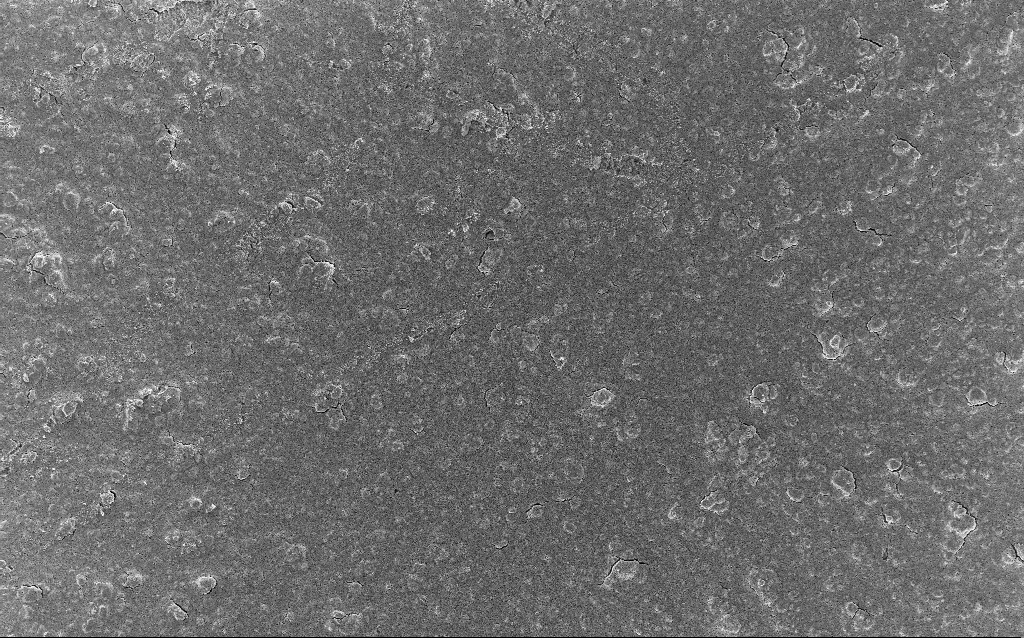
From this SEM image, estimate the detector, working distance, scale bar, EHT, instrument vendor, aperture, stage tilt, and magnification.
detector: InLens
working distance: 2.9 mm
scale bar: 20000 nm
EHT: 5 kV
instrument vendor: Zeiss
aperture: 30 µm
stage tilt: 0°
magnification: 0.77 K X